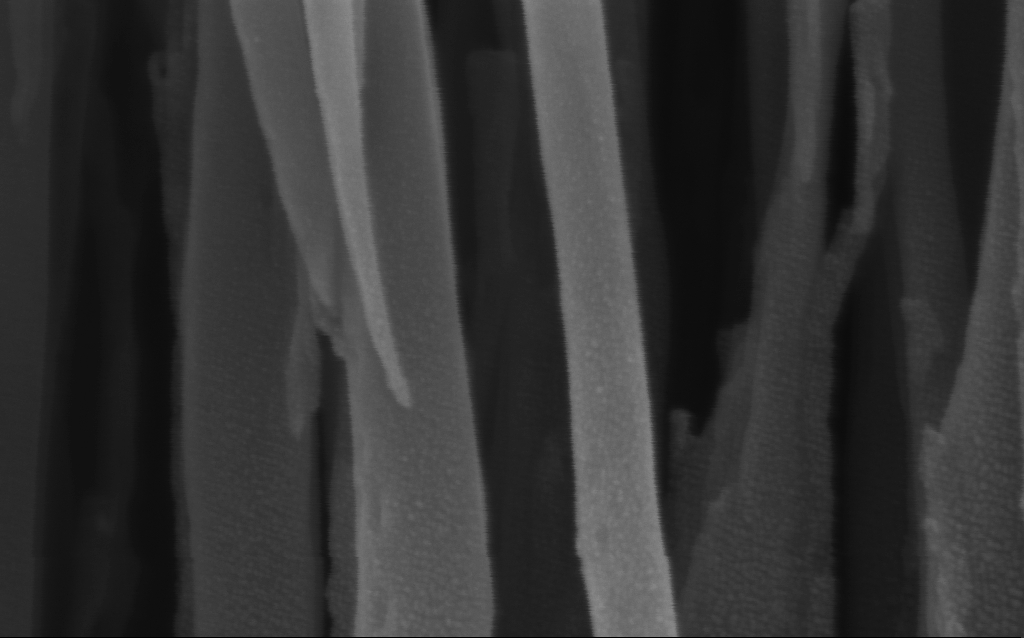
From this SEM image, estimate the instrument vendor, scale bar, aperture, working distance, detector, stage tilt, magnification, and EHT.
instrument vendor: Zeiss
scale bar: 100 nm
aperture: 30 µm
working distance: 3 mm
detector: InLens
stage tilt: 0°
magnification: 306.78 K X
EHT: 3 kV